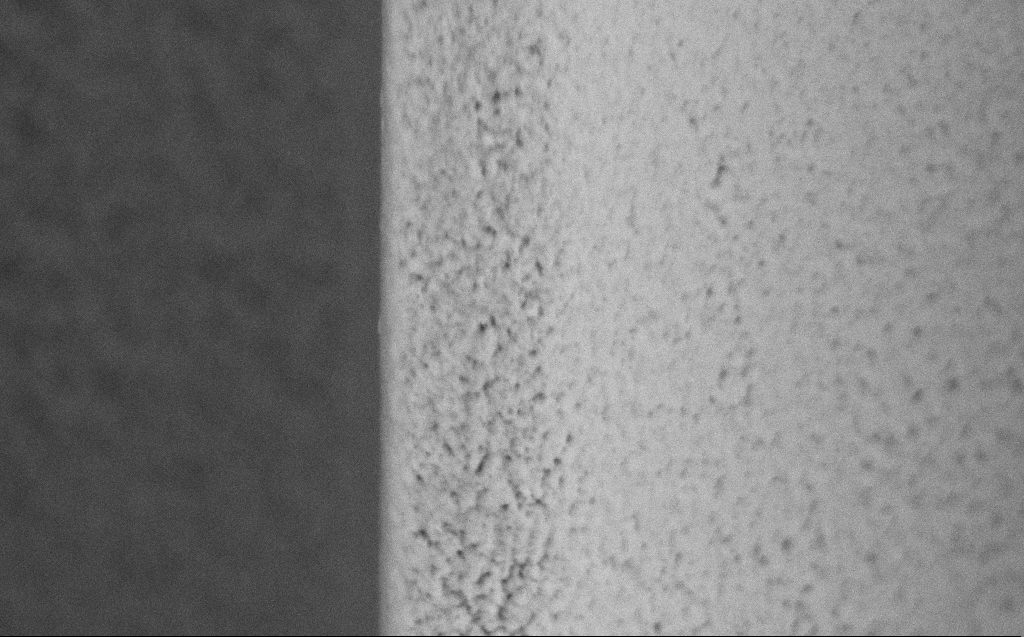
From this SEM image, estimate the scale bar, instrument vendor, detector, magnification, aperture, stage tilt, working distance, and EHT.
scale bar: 1000 nm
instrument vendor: Zeiss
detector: SE2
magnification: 64.38 K X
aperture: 30 µm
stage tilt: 45°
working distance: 5 mm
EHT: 5 kV